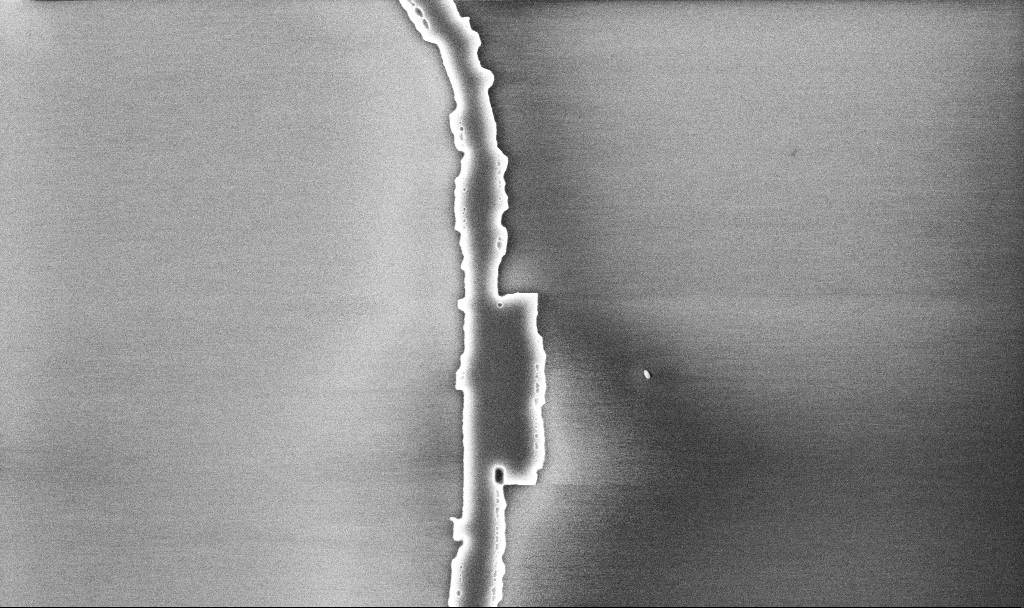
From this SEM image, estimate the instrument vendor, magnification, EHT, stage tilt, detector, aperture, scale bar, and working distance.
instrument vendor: Zeiss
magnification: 23.82 K X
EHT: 5 kV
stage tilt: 0°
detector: InLens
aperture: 30 µm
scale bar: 2000 nm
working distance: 10.1 mm